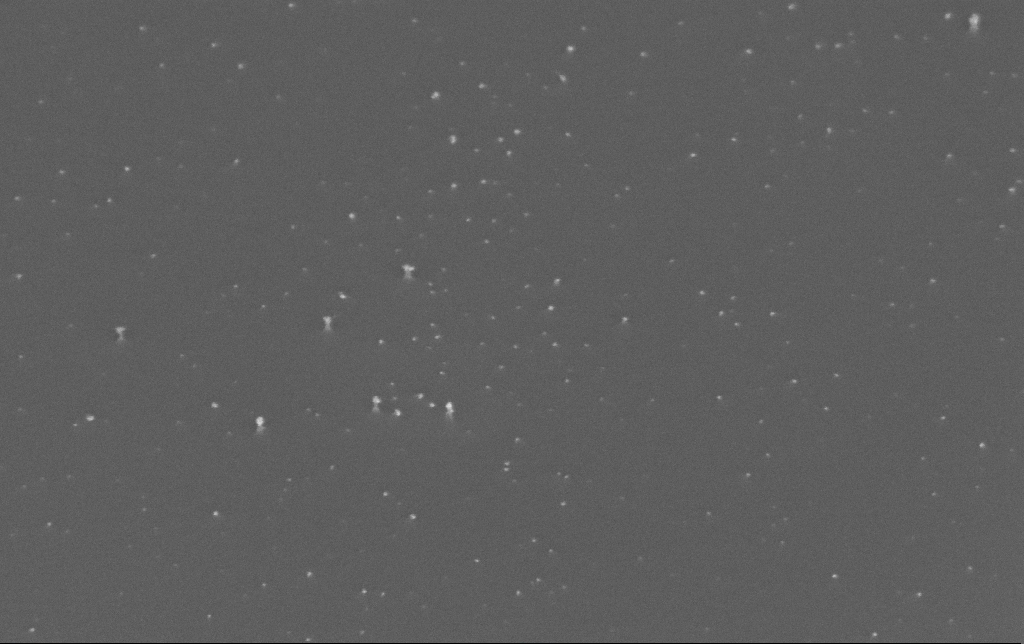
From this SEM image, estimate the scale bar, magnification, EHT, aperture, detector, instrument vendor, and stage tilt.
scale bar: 100 nm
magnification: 200 K X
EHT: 10 kV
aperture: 30 µm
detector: InLens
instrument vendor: Zeiss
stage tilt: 45°